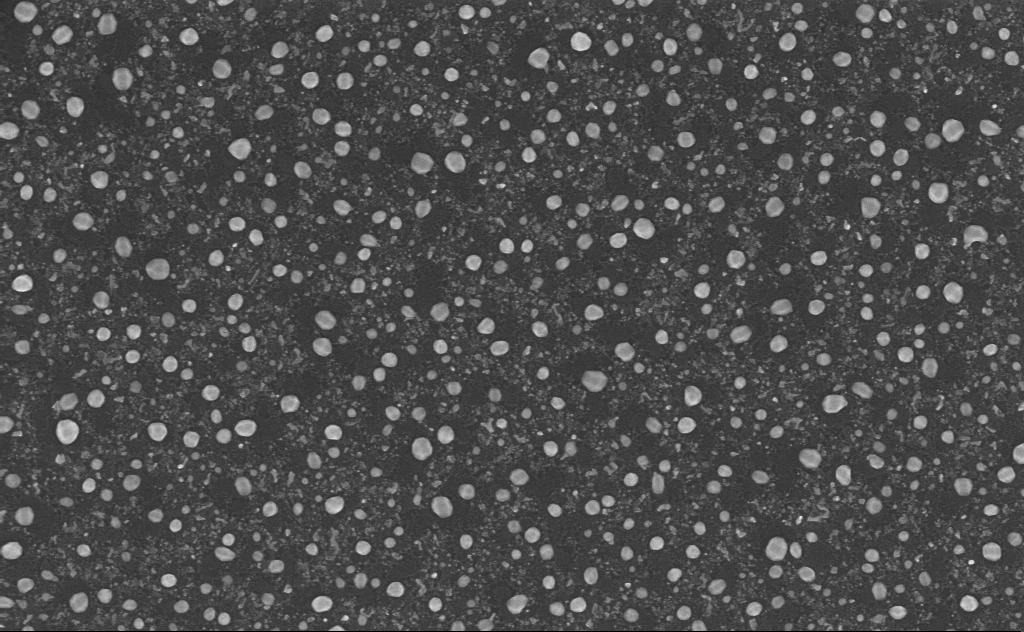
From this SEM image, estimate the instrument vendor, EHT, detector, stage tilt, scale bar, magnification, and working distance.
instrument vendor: Zeiss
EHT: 5 kV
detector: InLens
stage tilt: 0°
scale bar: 1000 nm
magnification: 60 K X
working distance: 4 mm